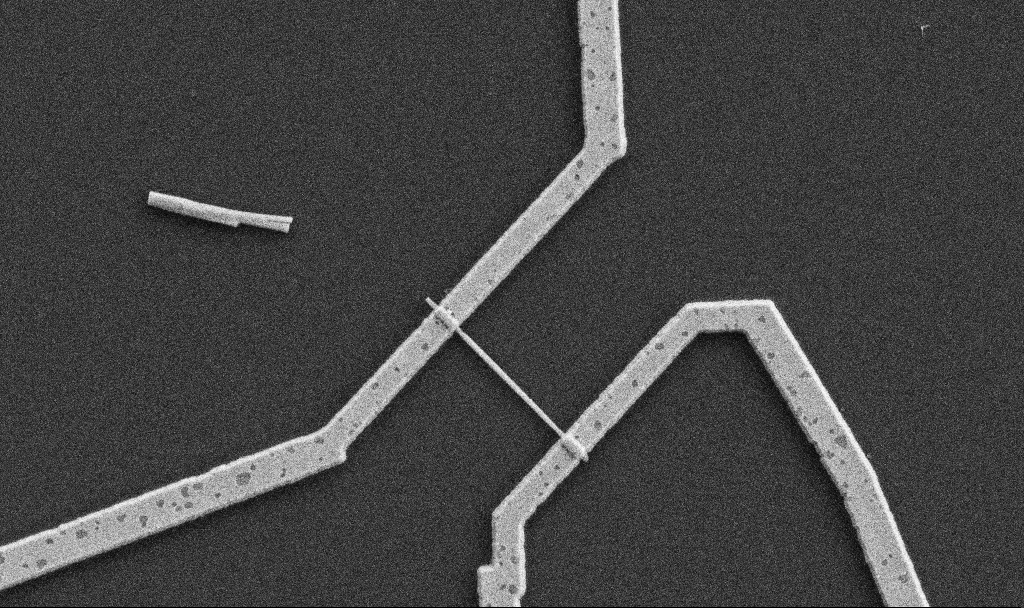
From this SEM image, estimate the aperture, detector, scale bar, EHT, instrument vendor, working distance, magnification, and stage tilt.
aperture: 30 µm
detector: SE2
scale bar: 1000 nm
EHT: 5 kV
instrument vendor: Zeiss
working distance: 10.7 mm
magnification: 20 K X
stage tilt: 0°